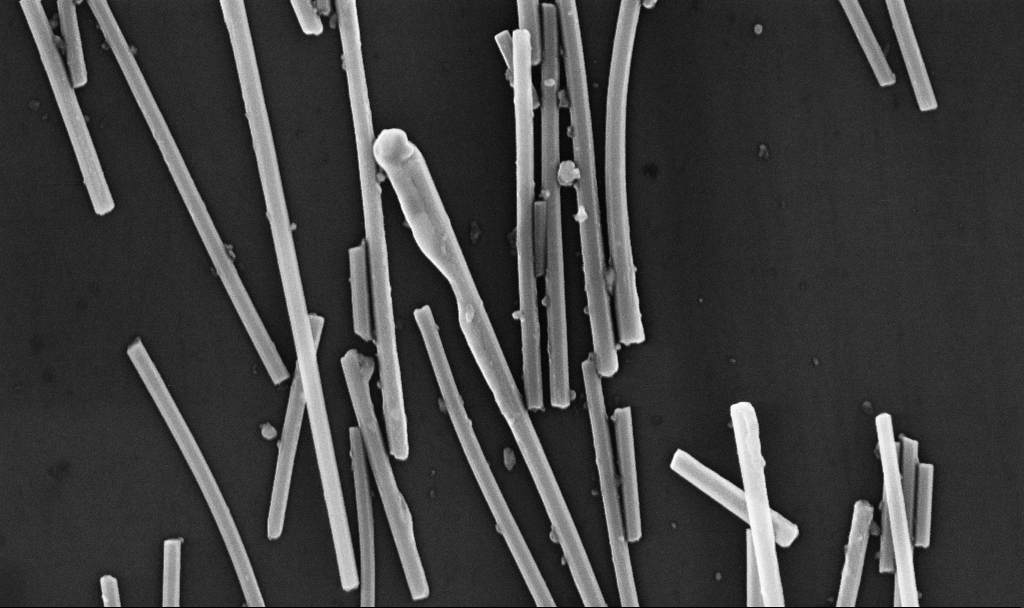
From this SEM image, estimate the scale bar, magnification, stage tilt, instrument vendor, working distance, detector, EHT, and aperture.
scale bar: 1000 nm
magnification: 61.34 K X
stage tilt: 0°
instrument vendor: Zeiss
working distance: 6.7 mm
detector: InLens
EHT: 10 kV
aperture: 30 µm